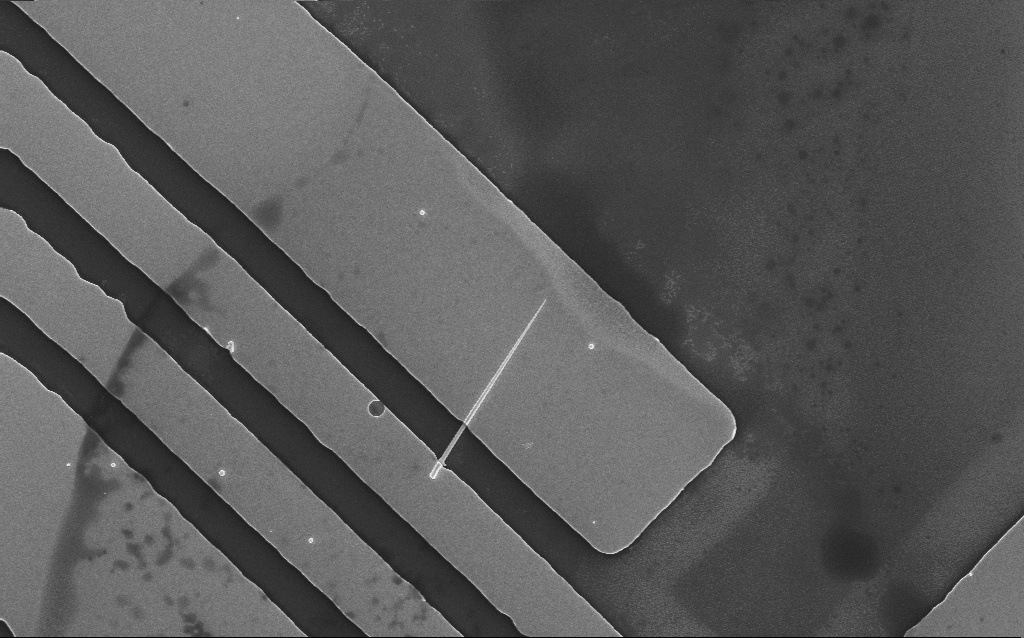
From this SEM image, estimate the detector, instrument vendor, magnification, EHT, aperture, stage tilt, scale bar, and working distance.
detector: InLens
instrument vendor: Zeiss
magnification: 6.76 K X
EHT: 10 kV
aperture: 30 µm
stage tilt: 0°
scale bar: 10000 nm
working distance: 4 mm